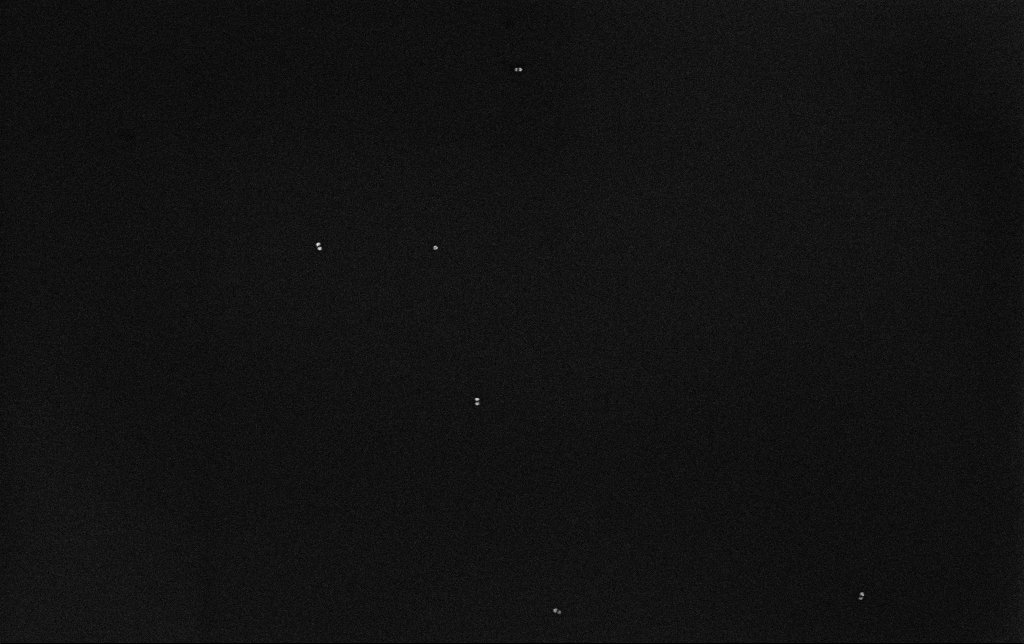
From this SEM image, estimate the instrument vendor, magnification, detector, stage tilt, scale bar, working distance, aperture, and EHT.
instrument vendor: Zeiss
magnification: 100 K X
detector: InLens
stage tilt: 0°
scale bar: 200 nm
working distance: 3.2 mm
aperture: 30 µm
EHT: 10 kV